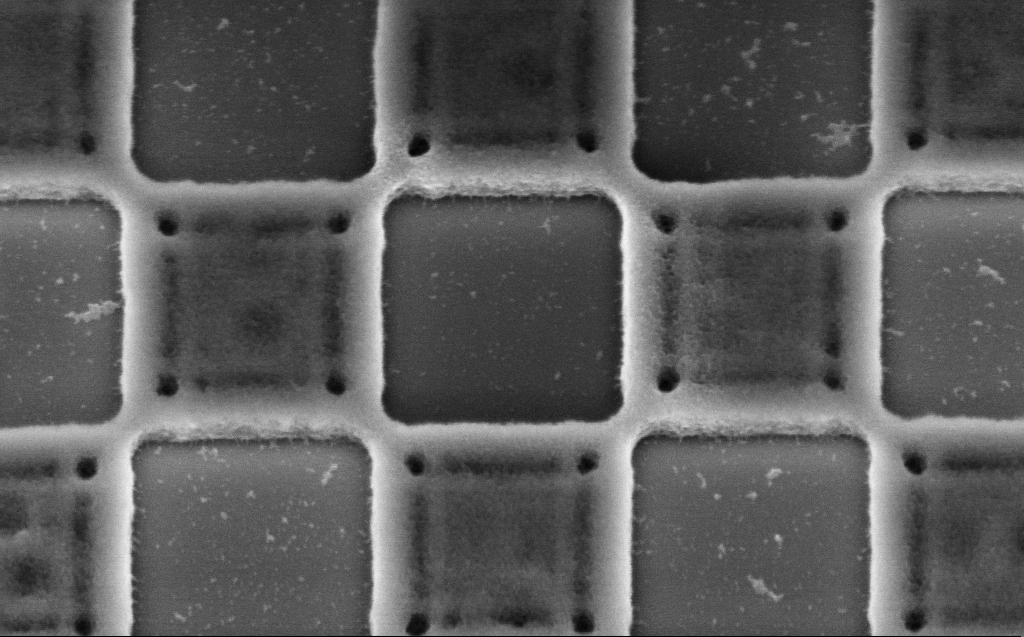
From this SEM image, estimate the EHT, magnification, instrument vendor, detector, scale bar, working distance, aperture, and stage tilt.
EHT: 3 kV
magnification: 92.41 K X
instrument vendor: Zeiss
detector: InLens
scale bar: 200 nm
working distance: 5 mm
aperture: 30 µm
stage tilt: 30°